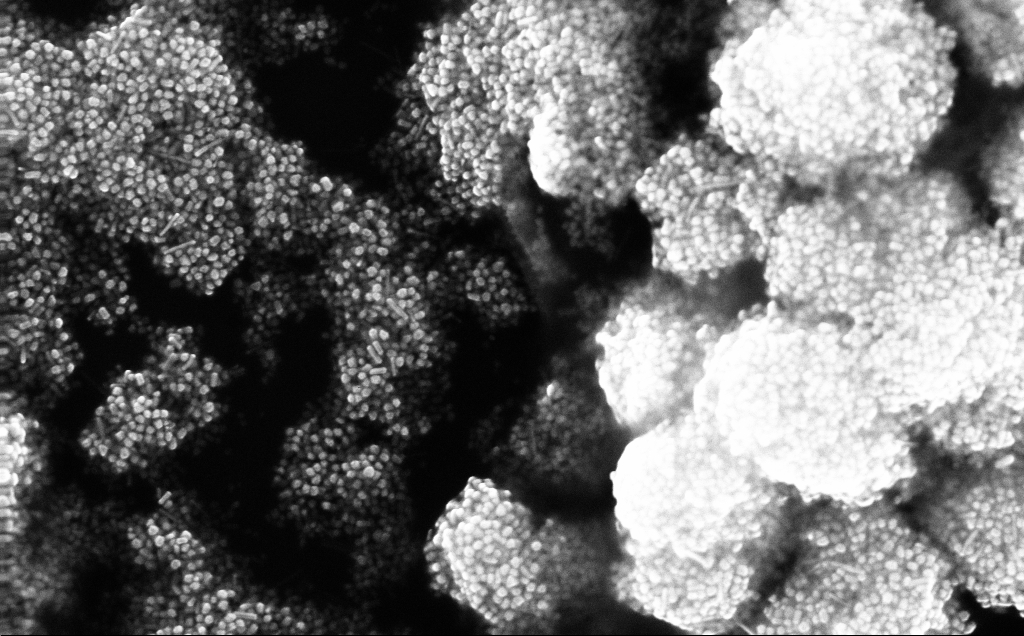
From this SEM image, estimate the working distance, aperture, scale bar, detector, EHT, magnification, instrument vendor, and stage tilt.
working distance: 3 mm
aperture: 30 µm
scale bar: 200 nm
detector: InLens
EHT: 20 kV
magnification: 95.86 K X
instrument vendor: Zeiss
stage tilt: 0°